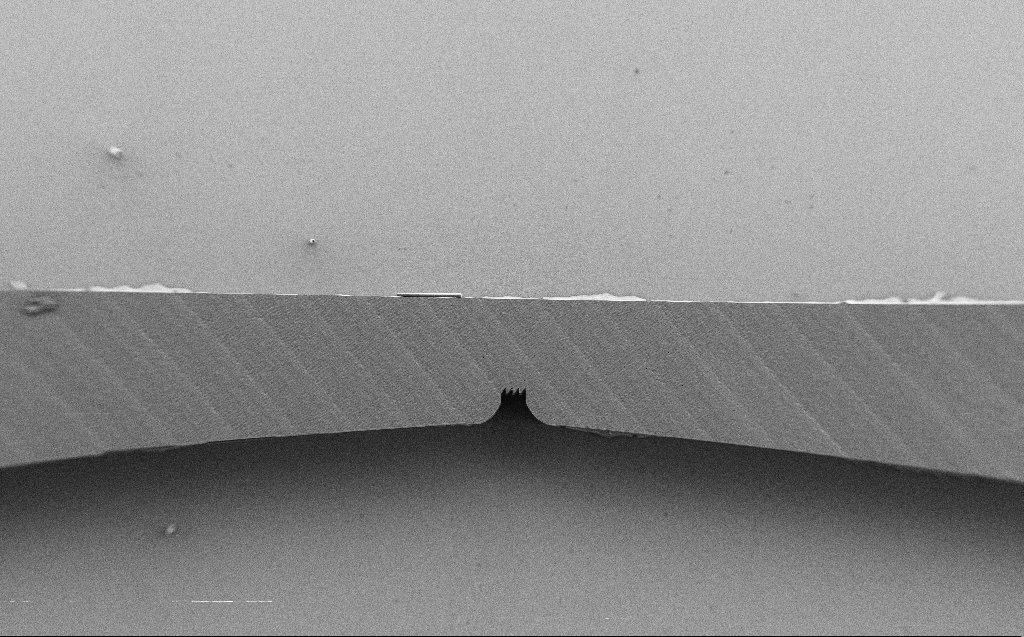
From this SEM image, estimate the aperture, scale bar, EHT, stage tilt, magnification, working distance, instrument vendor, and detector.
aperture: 30 µm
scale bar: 200000 nm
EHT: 1 kV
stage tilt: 0°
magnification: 0.217 K X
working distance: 6 mm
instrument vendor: Zeiss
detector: SE2